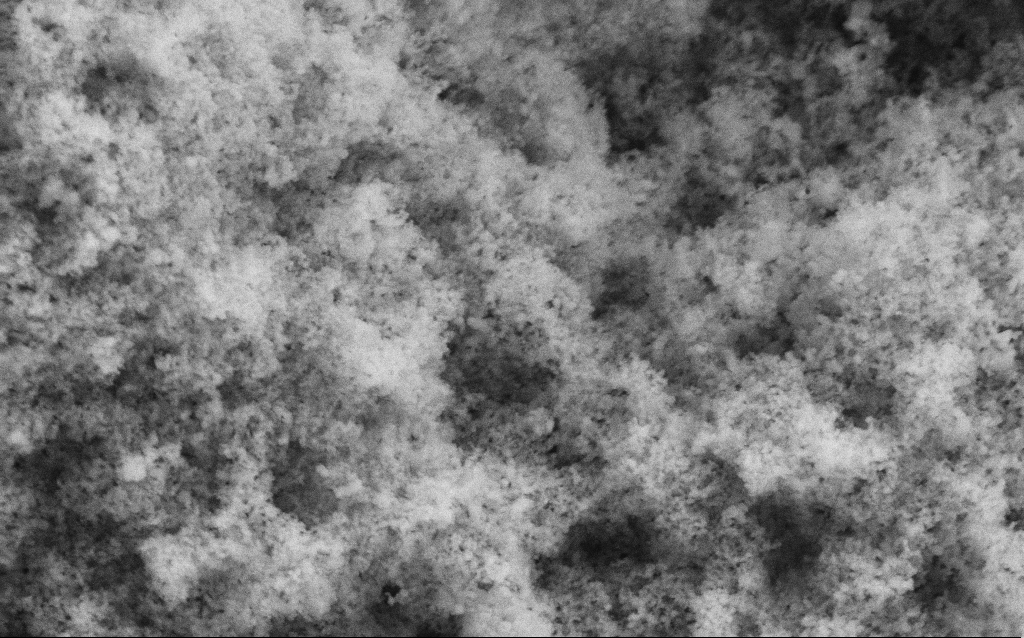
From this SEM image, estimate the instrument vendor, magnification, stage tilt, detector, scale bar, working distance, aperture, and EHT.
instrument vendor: Zeiss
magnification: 68.64 K X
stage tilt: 0°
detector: SE2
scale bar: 1000 nm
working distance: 4.1 mm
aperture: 30 µm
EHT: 5 kV